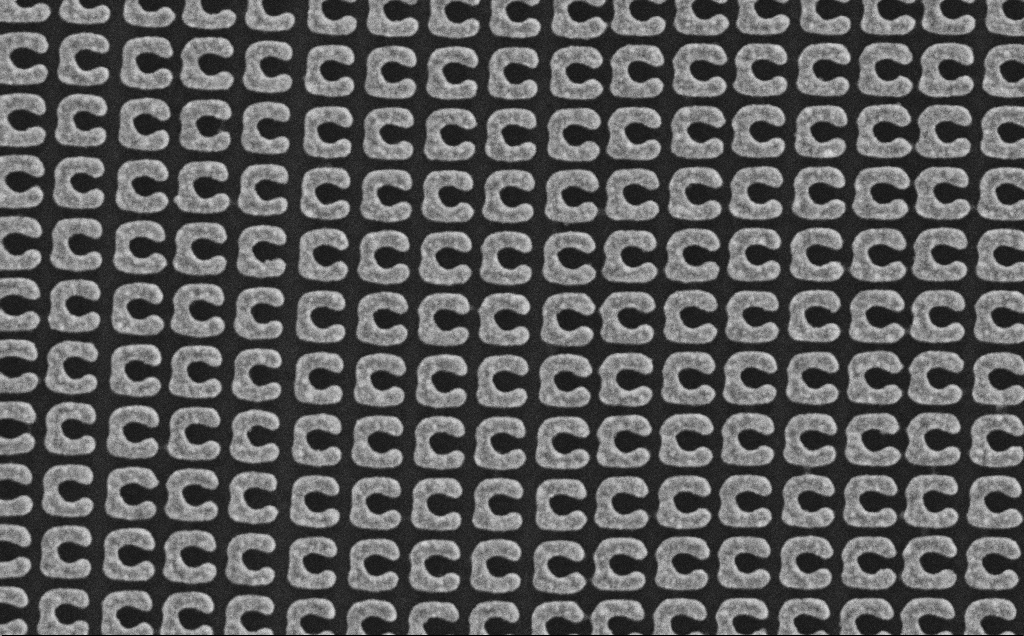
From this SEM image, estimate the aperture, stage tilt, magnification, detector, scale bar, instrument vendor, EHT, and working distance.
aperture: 30 µm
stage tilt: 0°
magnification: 49.2 K X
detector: SE2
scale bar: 1000 nm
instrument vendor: Zeiss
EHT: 5 kV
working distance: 2.3 mm